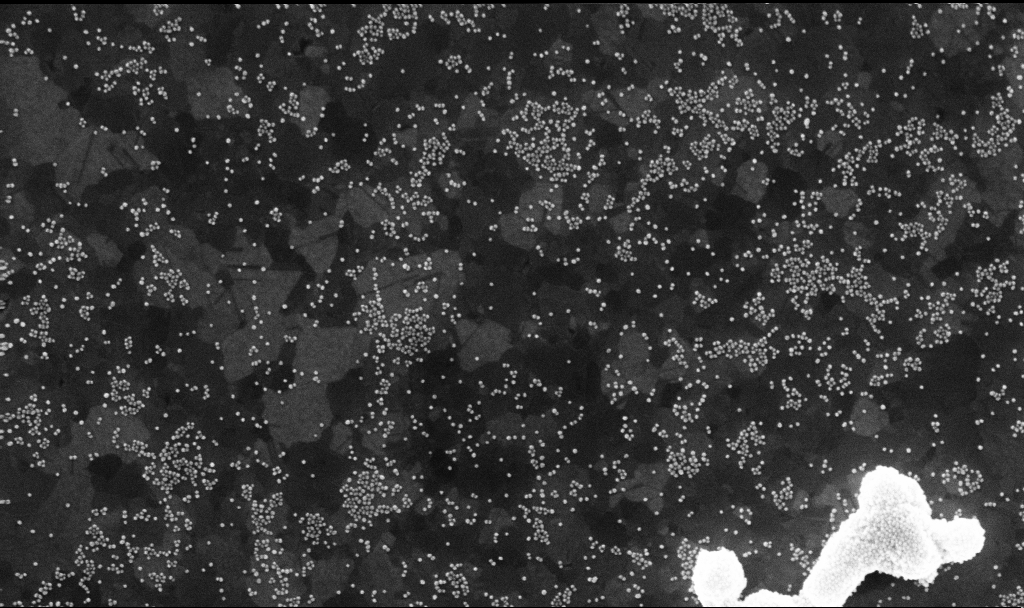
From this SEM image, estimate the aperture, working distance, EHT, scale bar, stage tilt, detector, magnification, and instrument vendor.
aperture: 30 µm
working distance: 3.8 mm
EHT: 10 kV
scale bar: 200 nm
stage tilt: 0°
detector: InLens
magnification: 80 K X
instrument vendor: Zeiss